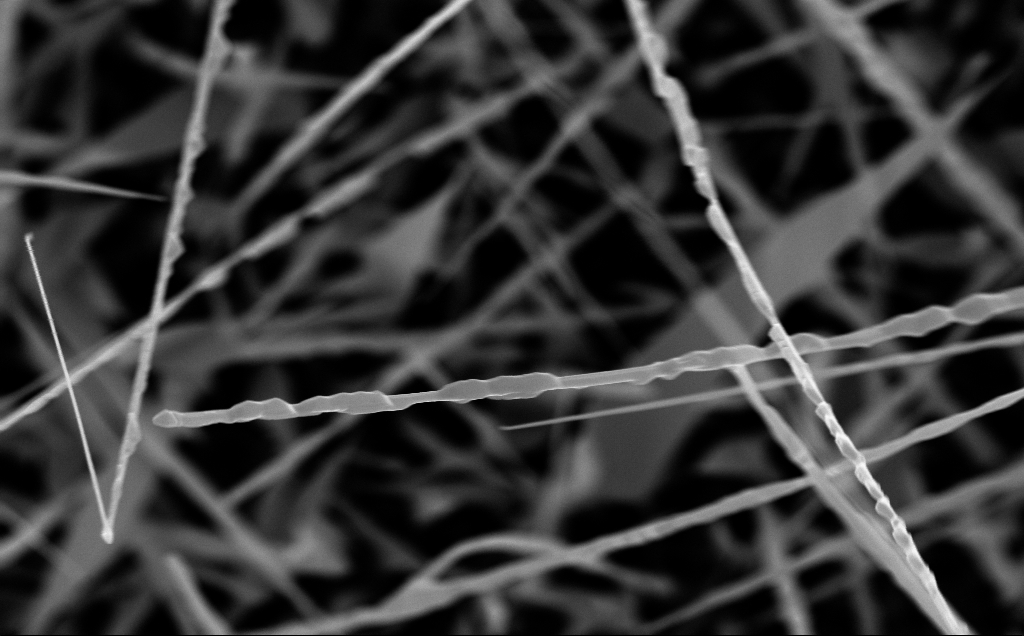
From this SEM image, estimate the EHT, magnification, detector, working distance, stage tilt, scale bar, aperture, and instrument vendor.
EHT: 10 kV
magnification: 38.11 K X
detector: InLens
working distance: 6 mm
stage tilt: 0°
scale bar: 1000 nm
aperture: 30 µm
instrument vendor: Zeiss